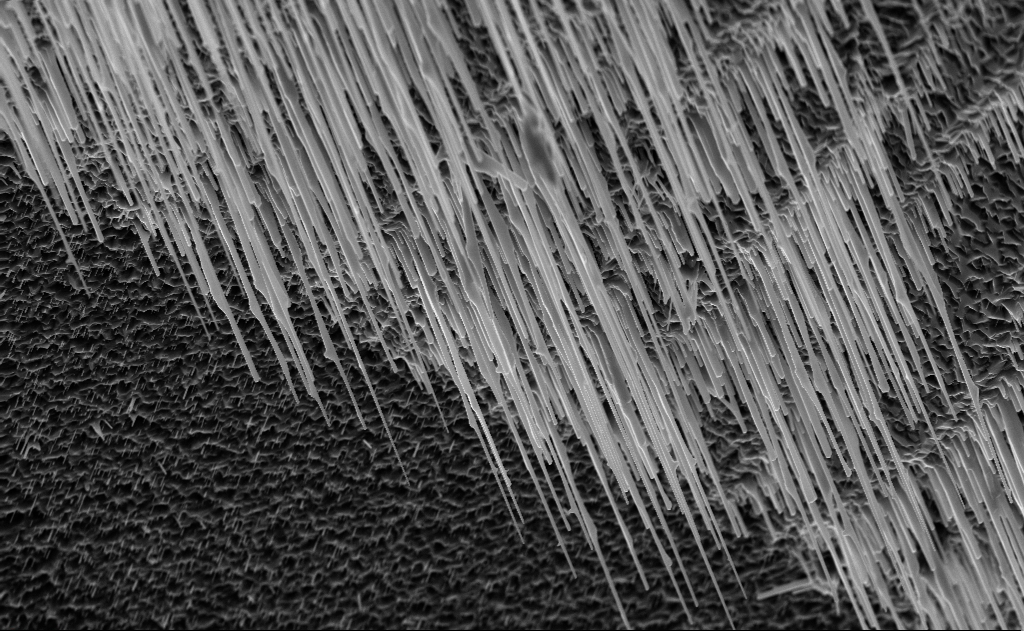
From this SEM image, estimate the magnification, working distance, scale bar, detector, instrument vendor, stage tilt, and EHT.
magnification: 10 K X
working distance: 6 mm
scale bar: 2000 nm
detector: InLens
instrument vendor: Zeiss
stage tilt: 0°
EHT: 10 kV